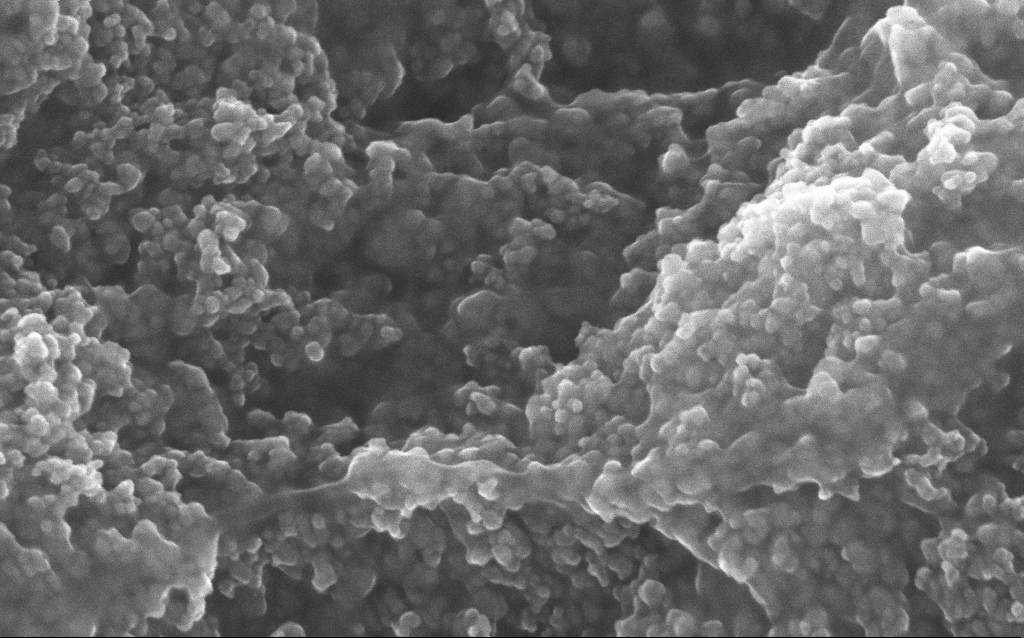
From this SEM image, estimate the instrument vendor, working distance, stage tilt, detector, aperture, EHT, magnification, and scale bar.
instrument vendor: Zeiss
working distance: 2.8 mm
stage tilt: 0°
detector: InLens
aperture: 30 µm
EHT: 10 kV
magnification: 204.13 K X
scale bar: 100 nm